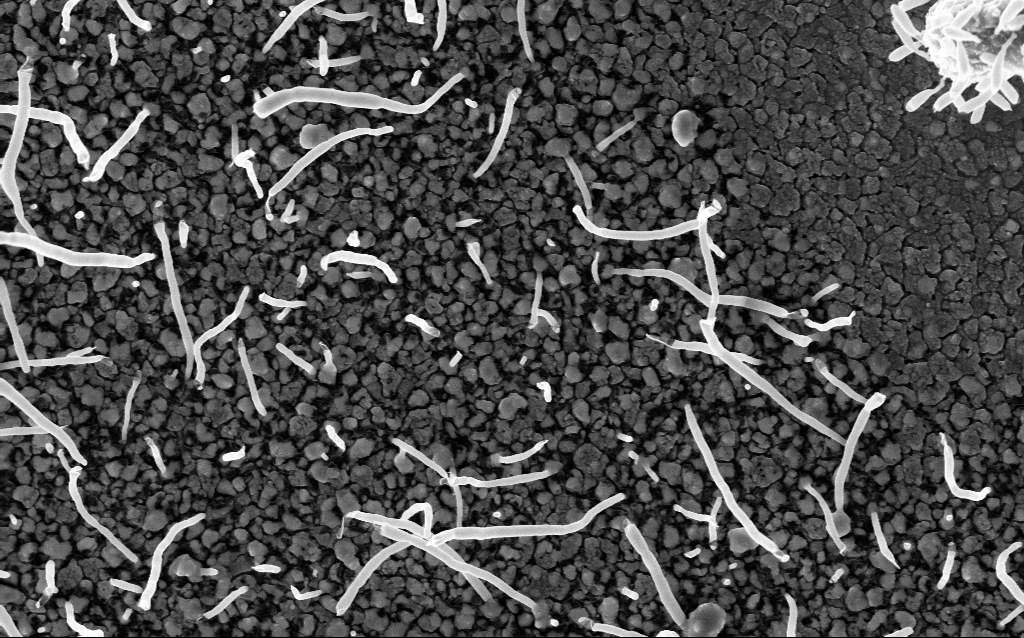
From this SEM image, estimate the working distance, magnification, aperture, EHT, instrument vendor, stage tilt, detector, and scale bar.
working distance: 2.2 mm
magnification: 50 K X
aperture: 30 µm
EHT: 5 kV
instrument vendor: Zeiss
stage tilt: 0°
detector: InLens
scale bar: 1000 nm